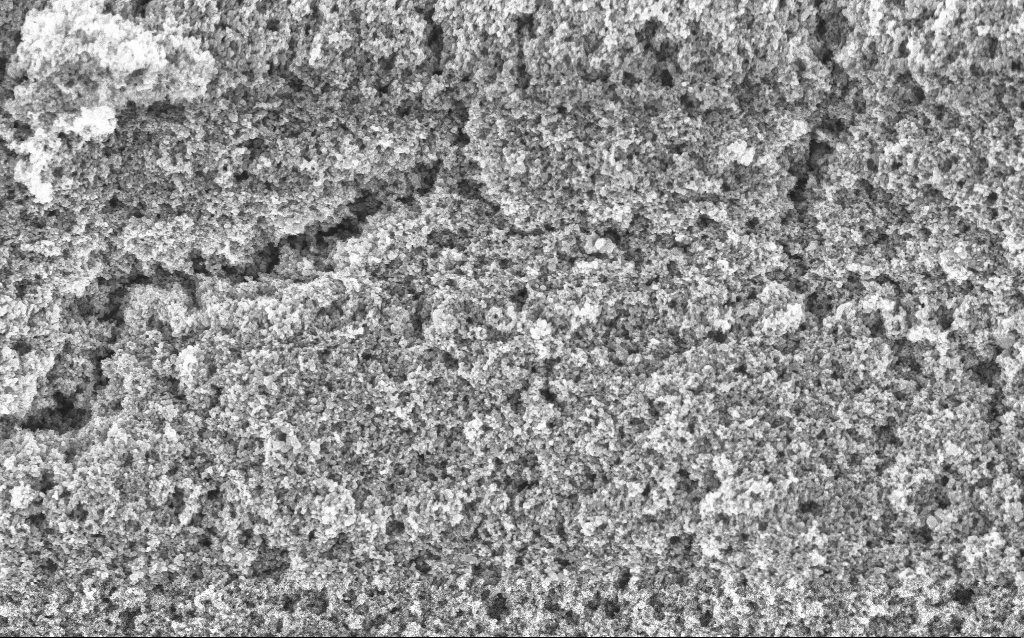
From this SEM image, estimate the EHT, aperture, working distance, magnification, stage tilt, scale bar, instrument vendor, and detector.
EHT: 5 kV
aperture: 30 µm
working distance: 4.4 mm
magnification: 37.88 K X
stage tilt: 0°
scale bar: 1000 nm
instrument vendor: Zeiss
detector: InLens